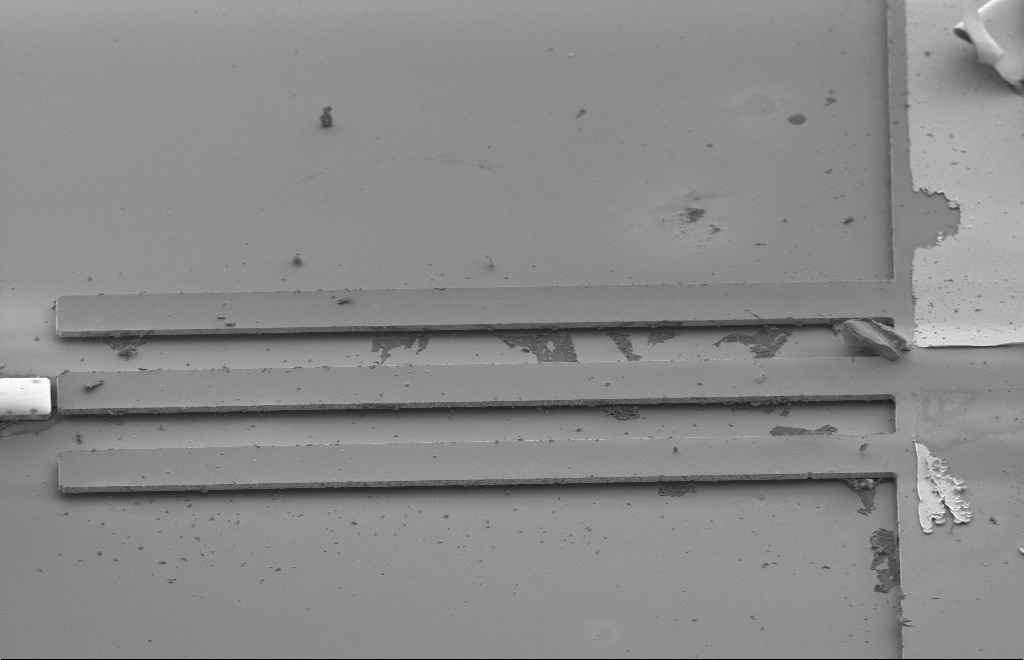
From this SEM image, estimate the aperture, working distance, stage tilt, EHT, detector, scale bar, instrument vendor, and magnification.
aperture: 30 µm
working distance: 13 mm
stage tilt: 47.2°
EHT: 2 kV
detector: SE2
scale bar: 10000 nm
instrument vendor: Zeiss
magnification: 1.1 K X